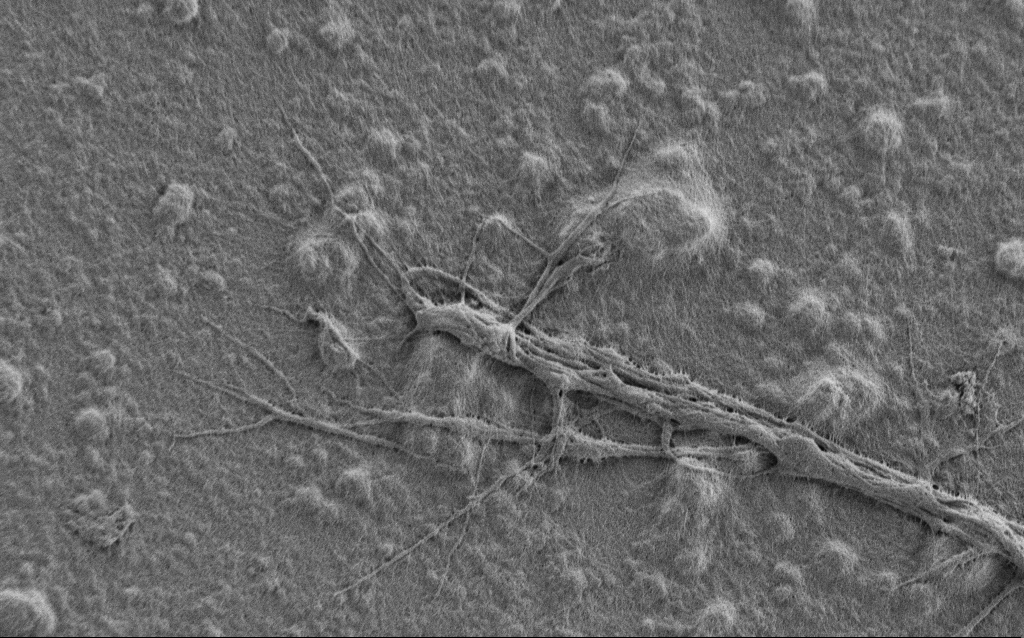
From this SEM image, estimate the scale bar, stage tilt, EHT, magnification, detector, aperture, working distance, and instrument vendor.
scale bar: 10000 nm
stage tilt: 0°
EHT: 0.9 kV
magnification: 7 K X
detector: SE2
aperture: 30 µm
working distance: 7 mm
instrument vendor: Zeiss